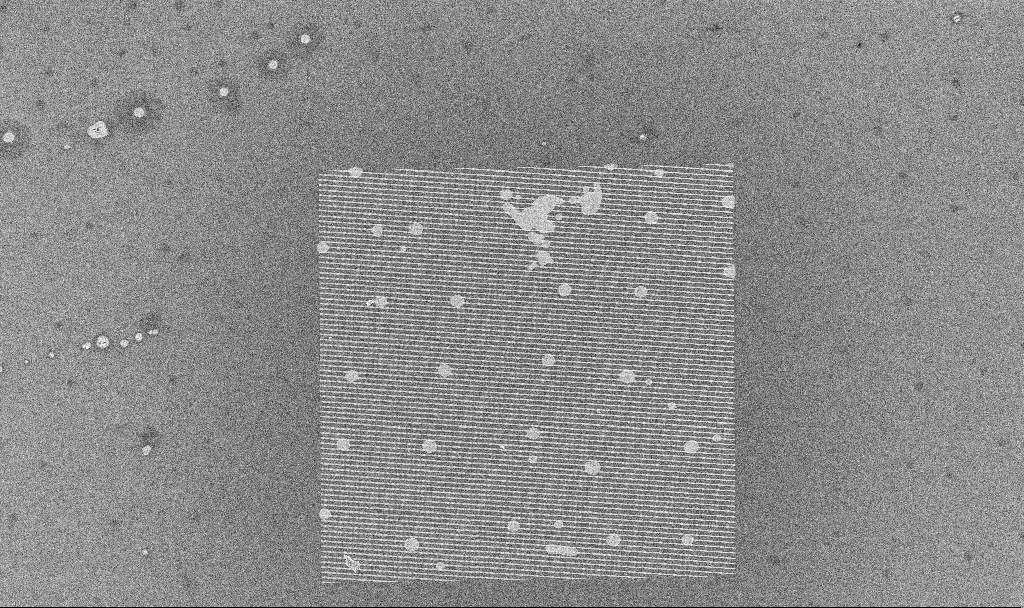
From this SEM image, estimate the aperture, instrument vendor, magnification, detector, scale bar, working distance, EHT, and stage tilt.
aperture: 30 µm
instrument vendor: Zeiss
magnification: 1.53 K X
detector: InLens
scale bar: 20000 nm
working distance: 4.6 mm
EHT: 5 kV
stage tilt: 0°